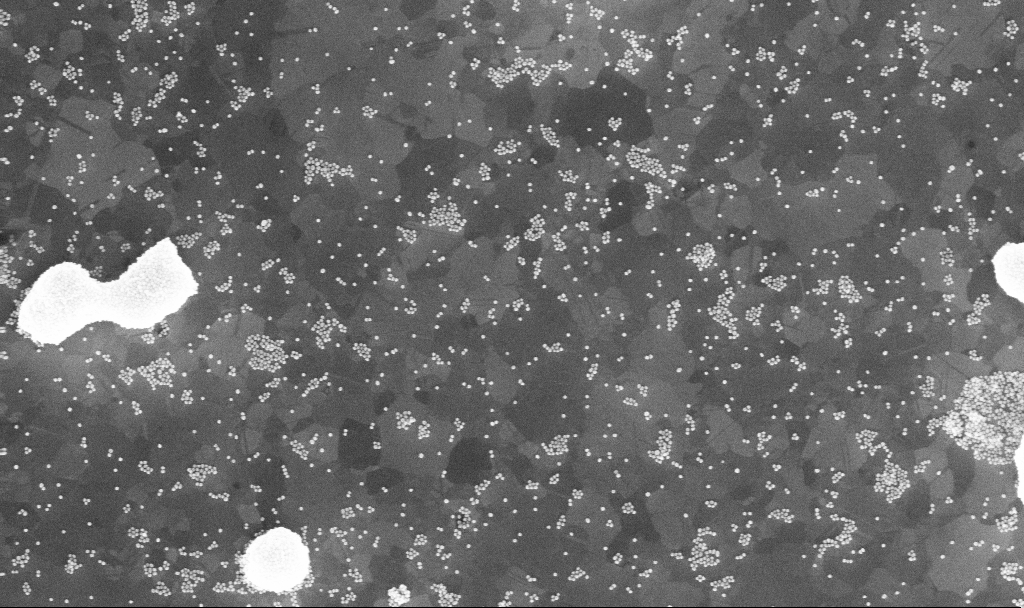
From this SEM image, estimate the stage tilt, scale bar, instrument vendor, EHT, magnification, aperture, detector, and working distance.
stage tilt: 0°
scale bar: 200 nm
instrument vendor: Zeiss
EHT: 10 kV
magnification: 80.58 K X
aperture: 30 µm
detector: InLens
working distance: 3.9 mm